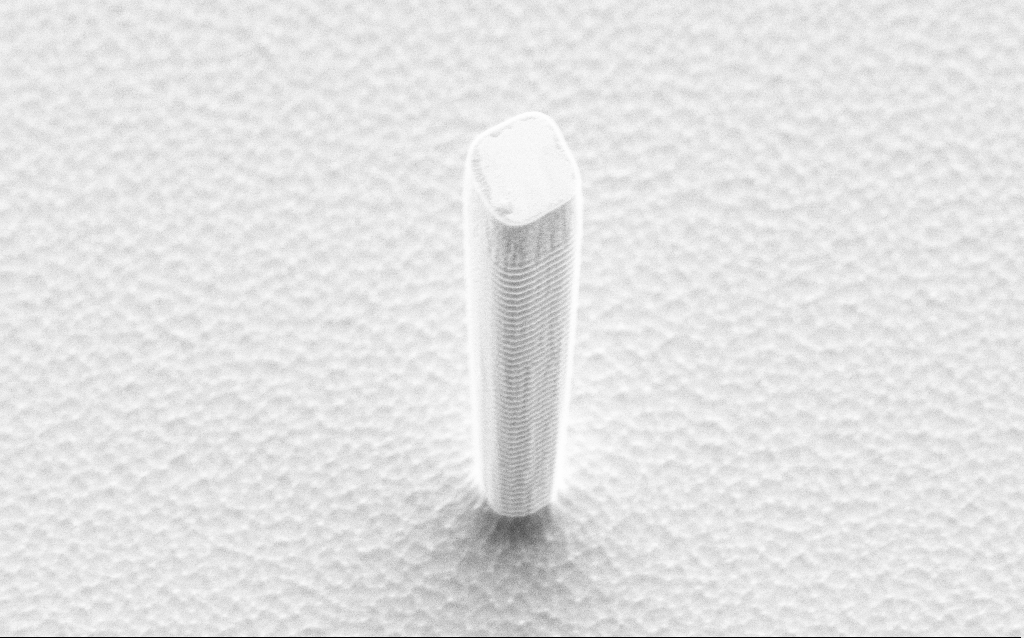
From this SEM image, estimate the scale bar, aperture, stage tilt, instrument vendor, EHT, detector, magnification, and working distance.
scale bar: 10000 nm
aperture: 30 µm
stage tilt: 50°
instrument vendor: Zeiss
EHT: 5 kV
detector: SE2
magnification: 6.19 K X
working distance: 10 mm